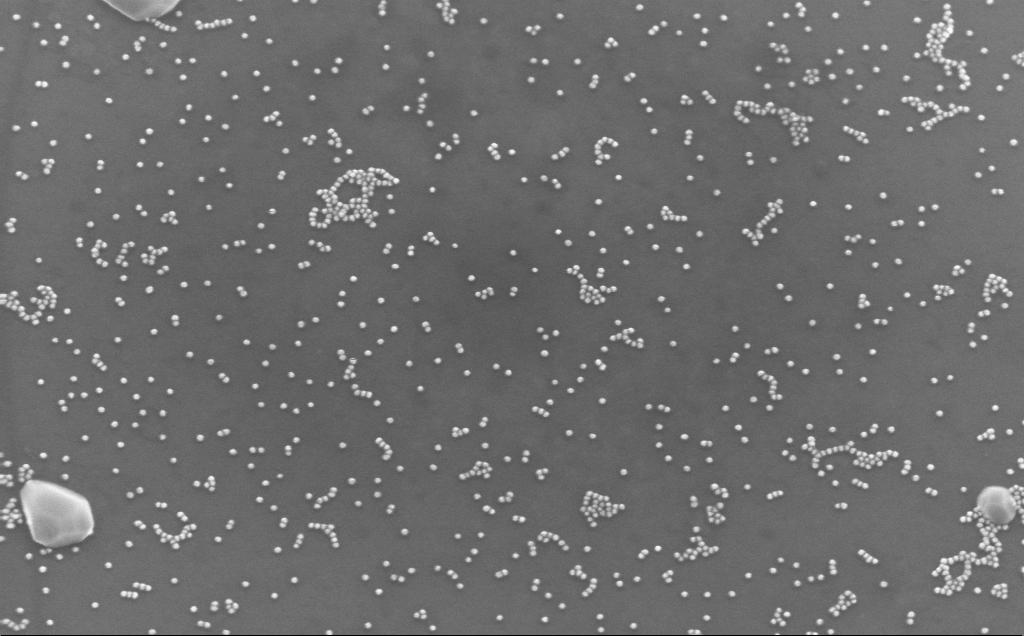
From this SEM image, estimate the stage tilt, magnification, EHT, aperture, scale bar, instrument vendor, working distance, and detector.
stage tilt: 25°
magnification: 124.8 K X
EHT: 10 kV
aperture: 30 µm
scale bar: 200 nm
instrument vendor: Zeiss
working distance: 4 mm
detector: InLens